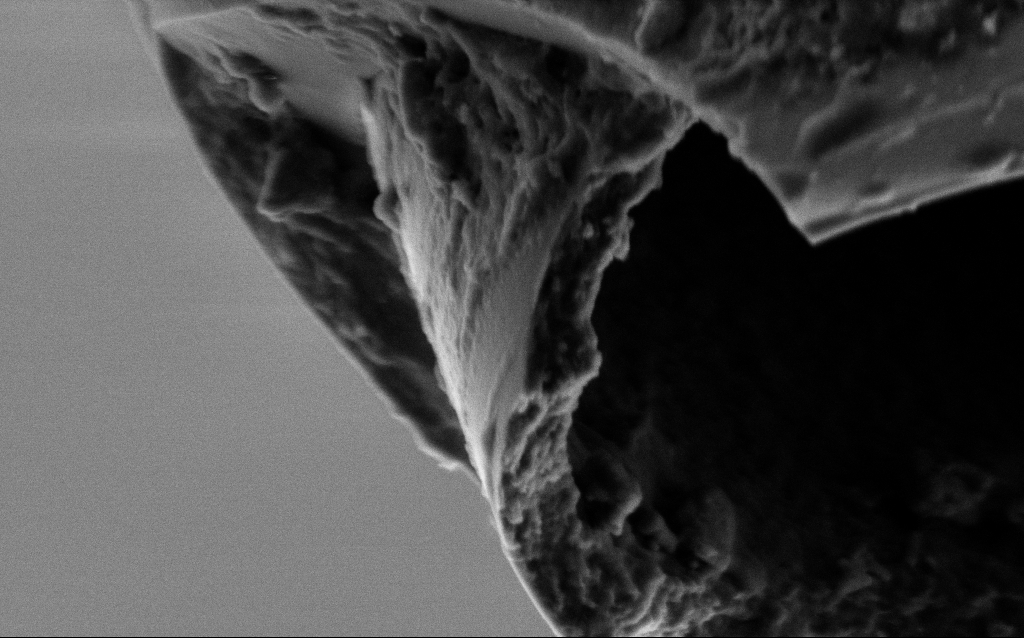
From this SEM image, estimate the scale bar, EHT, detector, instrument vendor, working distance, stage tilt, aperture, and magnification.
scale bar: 200 nm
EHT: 2 kV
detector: SE2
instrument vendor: Zeiss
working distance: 6 mm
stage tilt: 45°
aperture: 30 µm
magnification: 75 K X